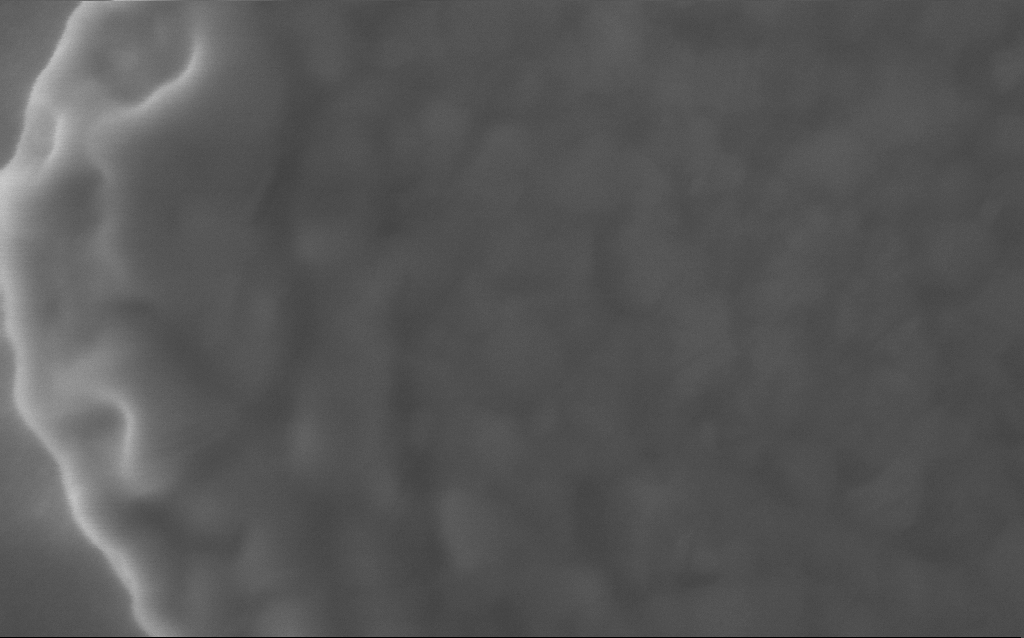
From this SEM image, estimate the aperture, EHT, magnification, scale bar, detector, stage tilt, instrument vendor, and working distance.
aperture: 30 µm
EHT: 5 kV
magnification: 293.18 K X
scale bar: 100 nm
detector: InLens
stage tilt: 0°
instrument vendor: Zeiss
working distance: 2 mm